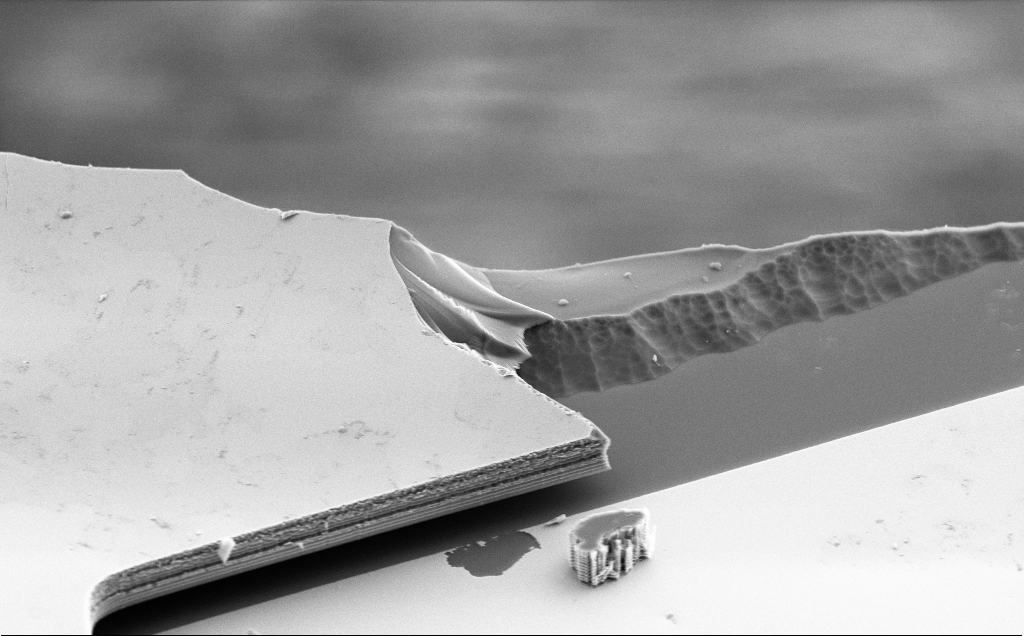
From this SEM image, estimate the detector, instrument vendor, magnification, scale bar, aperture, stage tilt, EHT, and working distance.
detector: SE2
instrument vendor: Zeiss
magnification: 7.31 K X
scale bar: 2000 nm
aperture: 30 µm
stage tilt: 50°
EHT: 5 kV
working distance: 10 mm